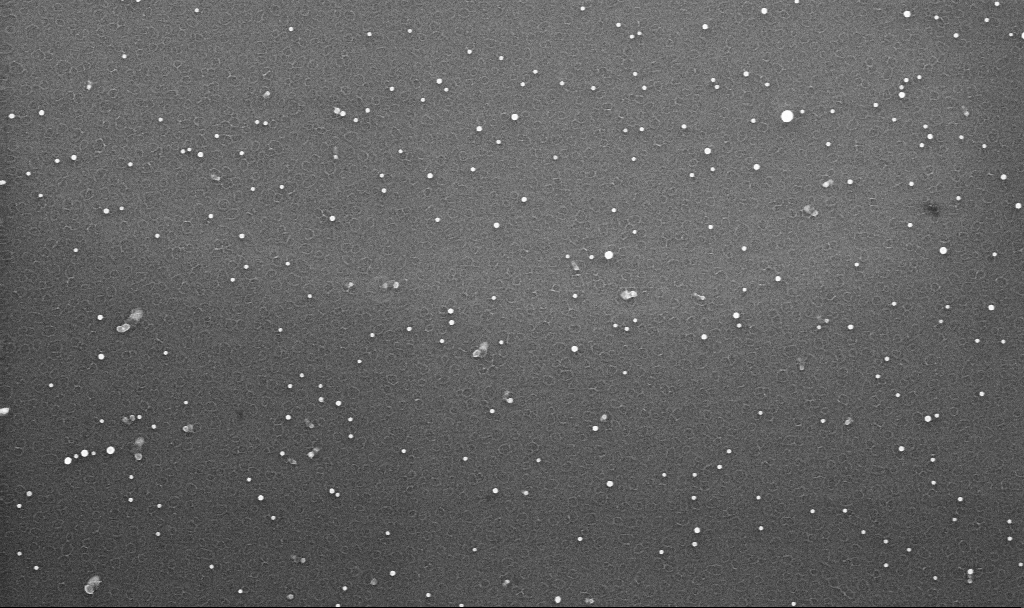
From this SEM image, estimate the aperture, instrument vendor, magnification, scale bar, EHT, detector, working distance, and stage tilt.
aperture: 30 µm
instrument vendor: Zeiss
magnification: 70 K X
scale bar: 1000 nm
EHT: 10 kV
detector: InLens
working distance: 3.3 mm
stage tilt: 0°